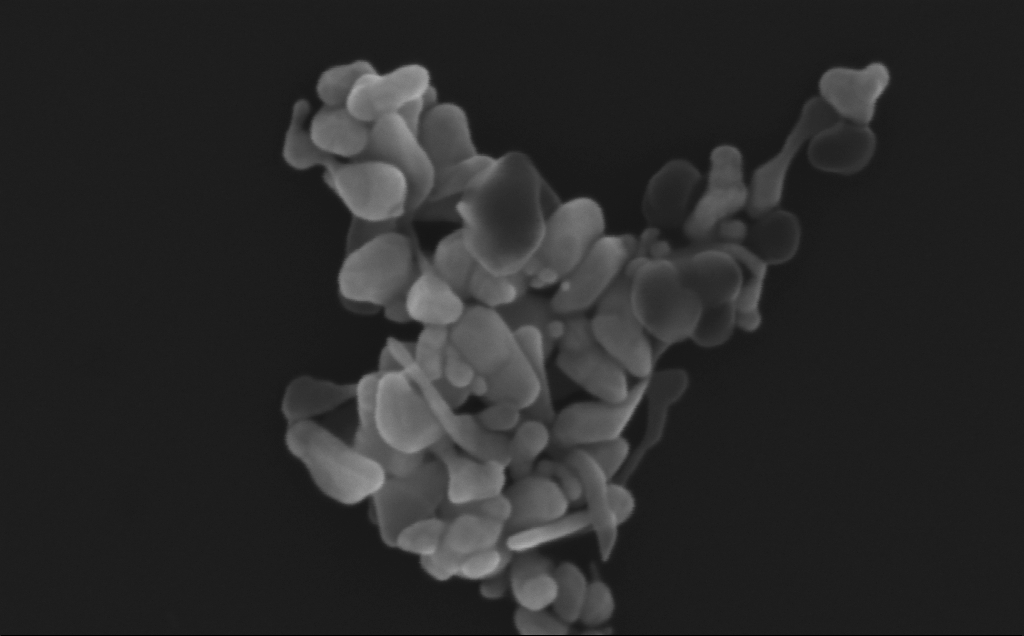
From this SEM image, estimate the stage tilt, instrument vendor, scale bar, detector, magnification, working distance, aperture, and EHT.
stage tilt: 0°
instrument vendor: Zeiss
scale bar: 200 nm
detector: InLens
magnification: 318.4 K X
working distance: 3 mm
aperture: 30 µm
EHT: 10 kV